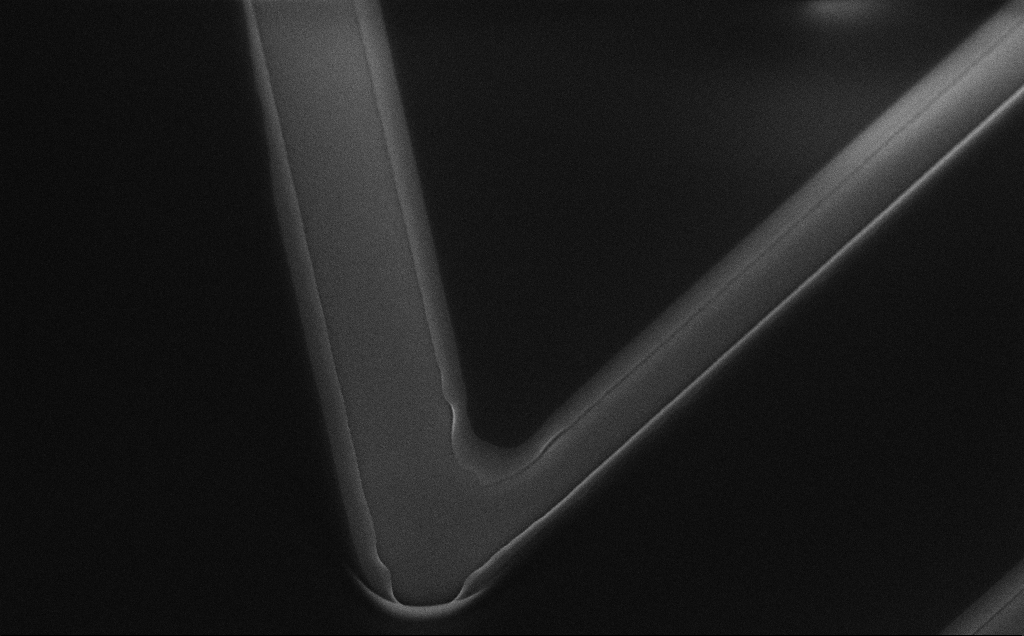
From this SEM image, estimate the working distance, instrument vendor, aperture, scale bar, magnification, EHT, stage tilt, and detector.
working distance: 11 mm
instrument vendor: Zeiss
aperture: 30 µm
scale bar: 2000 nm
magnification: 7.91 K X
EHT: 10 kV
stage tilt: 50°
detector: InLens